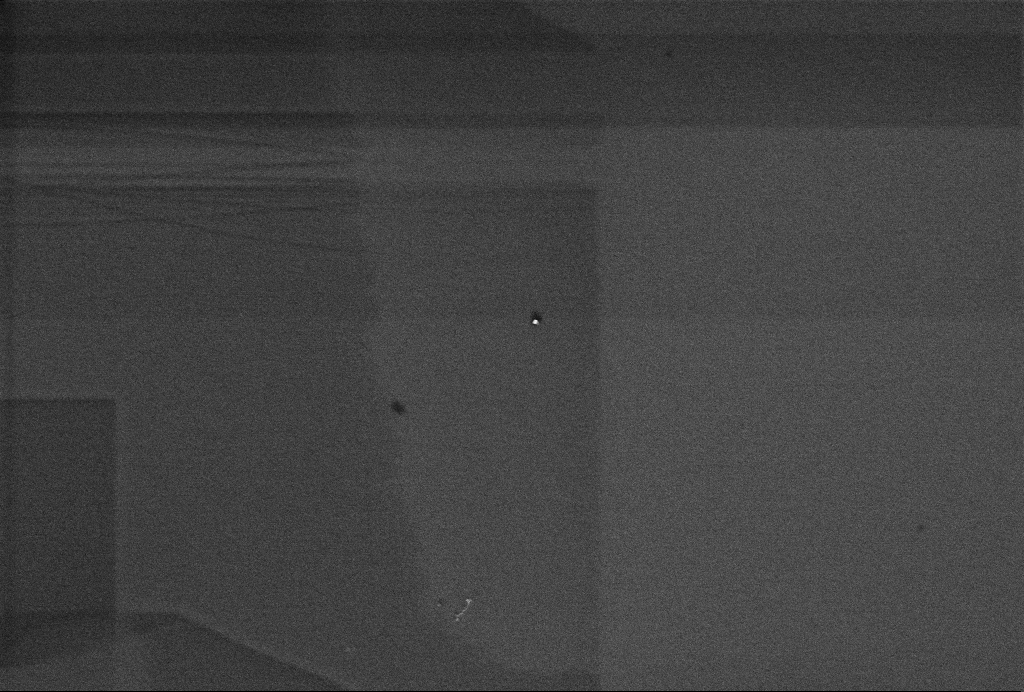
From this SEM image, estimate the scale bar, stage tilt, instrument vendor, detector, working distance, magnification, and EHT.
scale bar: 1000 nm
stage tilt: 0°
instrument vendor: Zeiss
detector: InLens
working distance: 3.3 mm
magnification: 60 K X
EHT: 1 kV